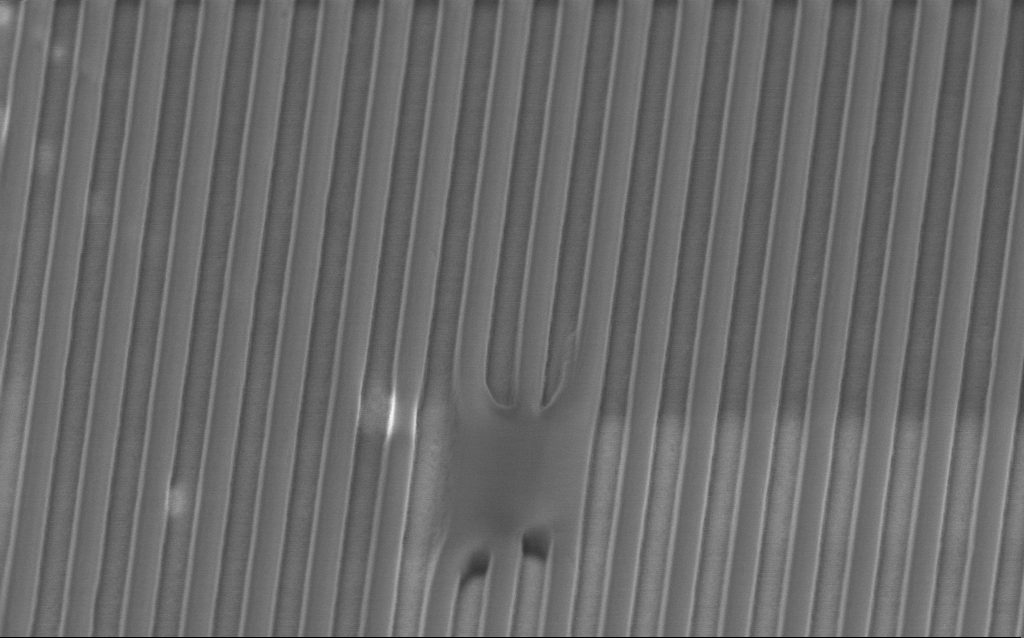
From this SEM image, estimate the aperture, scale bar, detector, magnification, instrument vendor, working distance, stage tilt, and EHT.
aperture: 30 µm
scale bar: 1000 nm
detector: InLens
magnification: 43.74 K X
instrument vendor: Zeiss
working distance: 3.6 mm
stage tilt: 45°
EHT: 2 kV